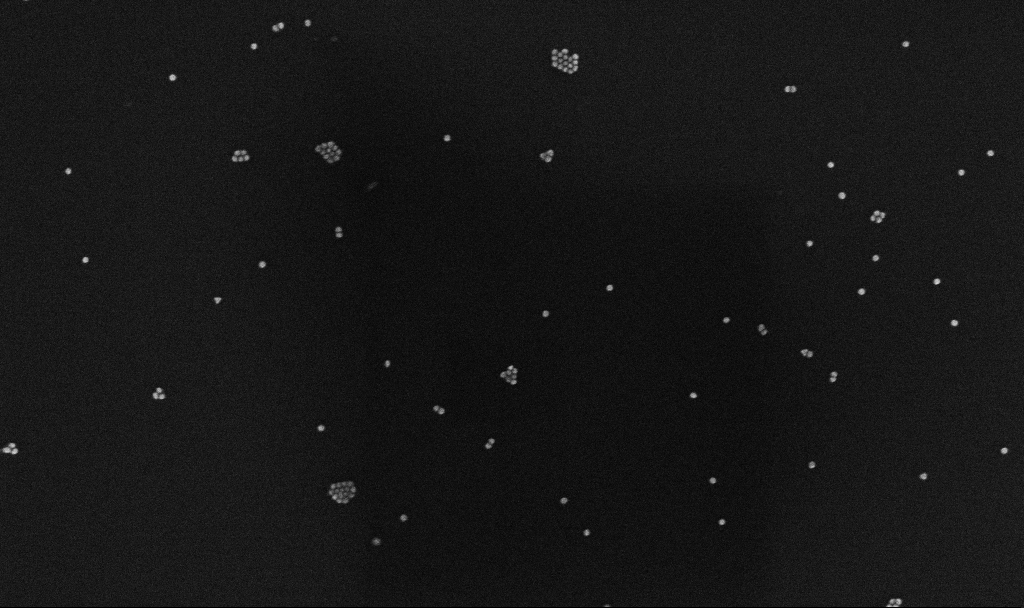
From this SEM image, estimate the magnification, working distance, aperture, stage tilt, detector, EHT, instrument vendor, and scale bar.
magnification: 100 K X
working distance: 3.3 mm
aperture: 30 µm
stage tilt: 0°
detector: InLens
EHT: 10 kV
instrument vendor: Zeiss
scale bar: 200 nm